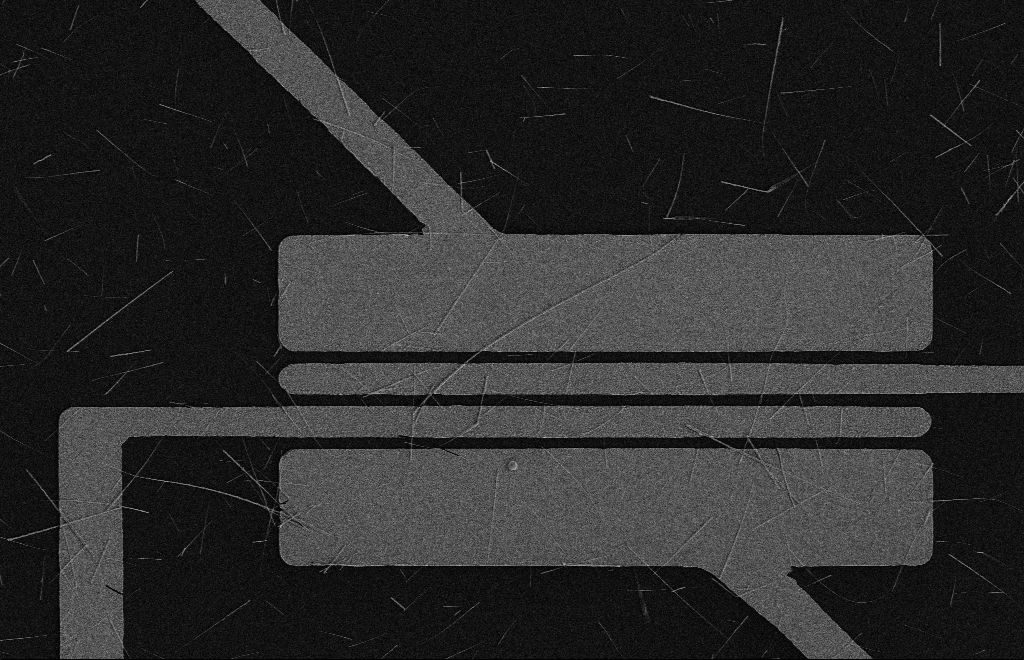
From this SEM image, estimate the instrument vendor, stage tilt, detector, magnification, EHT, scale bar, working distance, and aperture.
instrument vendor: Zeiss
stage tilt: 0°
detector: SE2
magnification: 3.96 K X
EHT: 5 kV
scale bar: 10000 nm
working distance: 16 mm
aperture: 10 µm